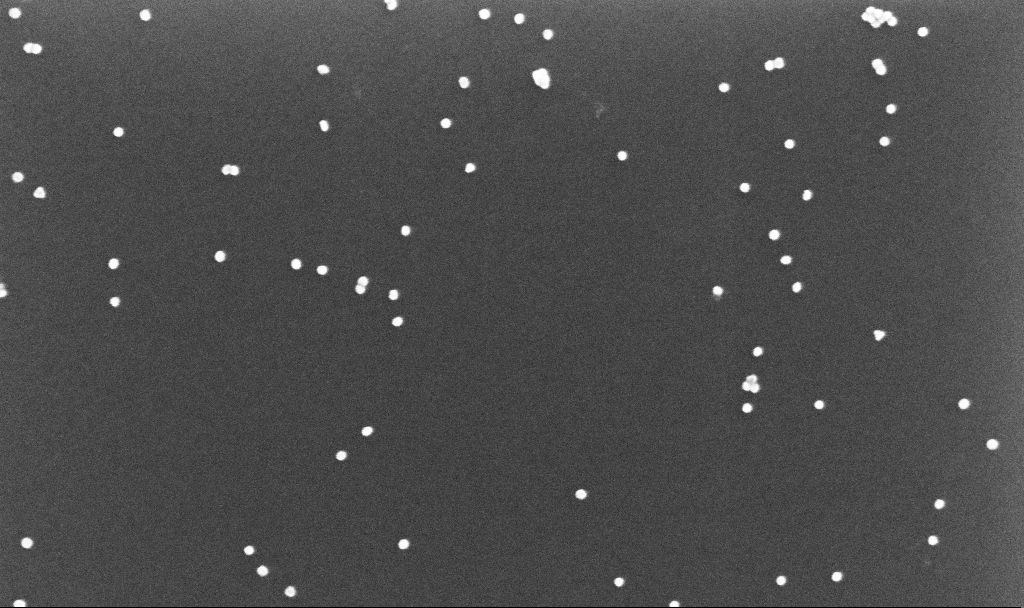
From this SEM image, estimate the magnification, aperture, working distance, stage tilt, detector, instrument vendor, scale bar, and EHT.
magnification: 150 K X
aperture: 30 µm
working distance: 3.4 mm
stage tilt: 0°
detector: InLens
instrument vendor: Zeiss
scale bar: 200 nm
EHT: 10 kV